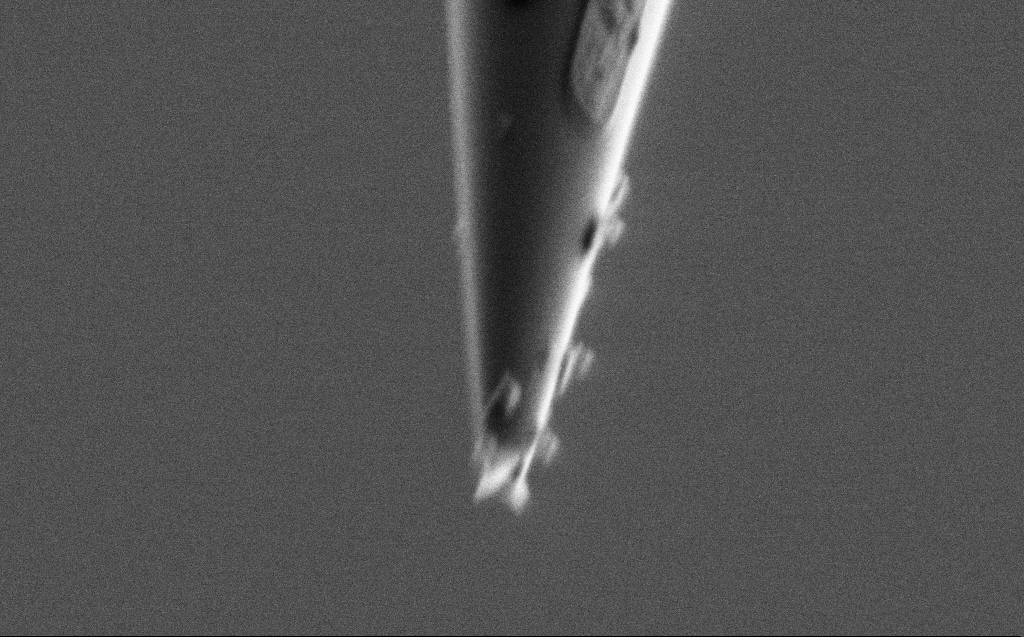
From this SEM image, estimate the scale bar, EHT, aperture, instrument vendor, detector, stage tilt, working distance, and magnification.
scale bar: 200 nm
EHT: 1 kV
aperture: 30 µm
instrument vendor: Zeiss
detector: SE2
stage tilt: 45°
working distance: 4 mm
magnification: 86.18 K X